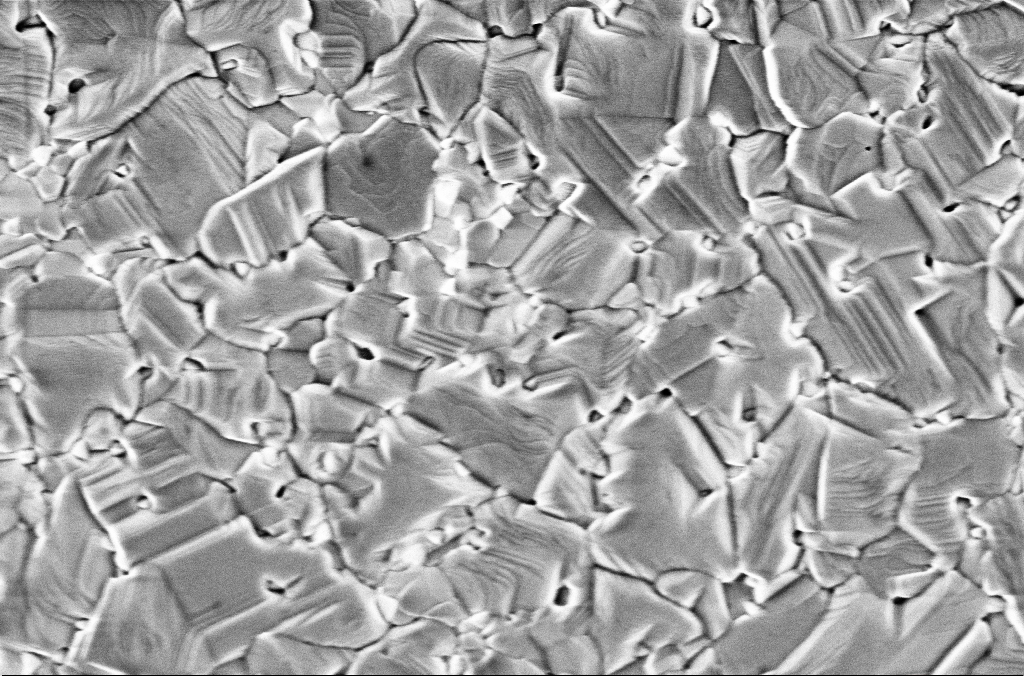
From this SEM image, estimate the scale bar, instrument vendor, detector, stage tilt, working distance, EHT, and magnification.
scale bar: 200 nm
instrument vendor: Zeiss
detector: InLens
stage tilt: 0°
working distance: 3 mm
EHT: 2 kV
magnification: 70 K X